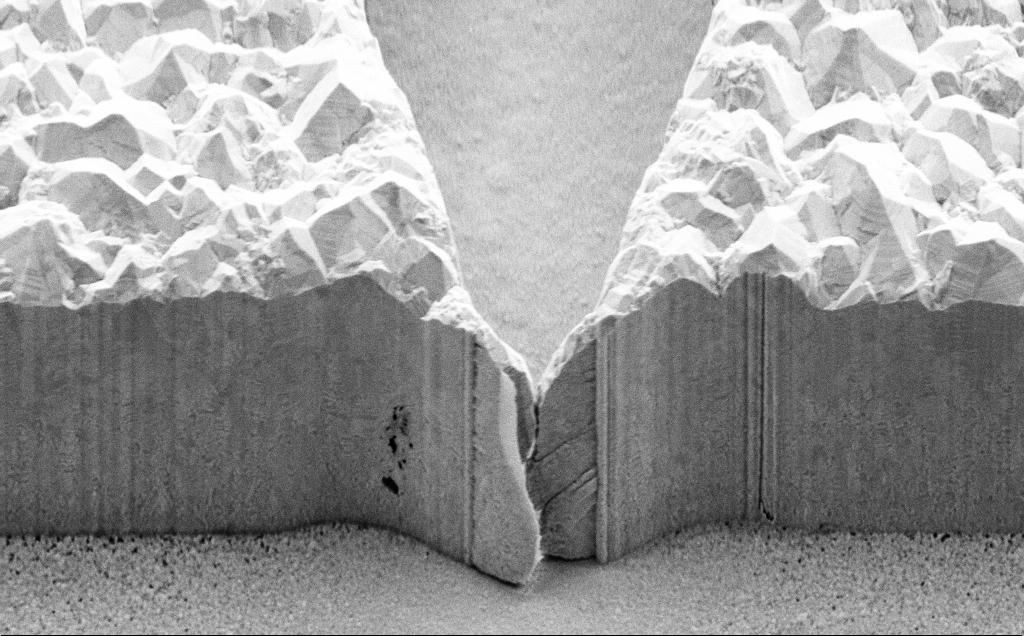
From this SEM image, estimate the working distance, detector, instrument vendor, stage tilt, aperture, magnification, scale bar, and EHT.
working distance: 9 mm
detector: SE2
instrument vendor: Zeiss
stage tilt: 45°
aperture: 30 µm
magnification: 13.11 K X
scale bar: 2000 nm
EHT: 5 kV